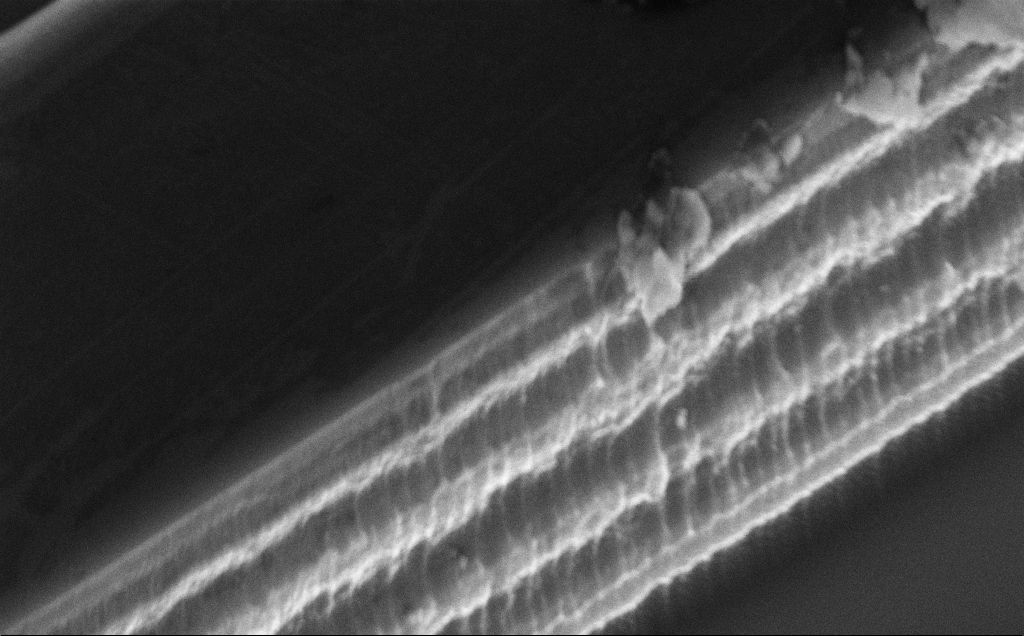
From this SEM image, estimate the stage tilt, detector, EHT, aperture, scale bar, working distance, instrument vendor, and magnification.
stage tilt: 50°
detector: InLens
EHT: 10 kV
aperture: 30 µm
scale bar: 2000 nm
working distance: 10 mm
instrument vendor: Zeiss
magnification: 23.08 K X